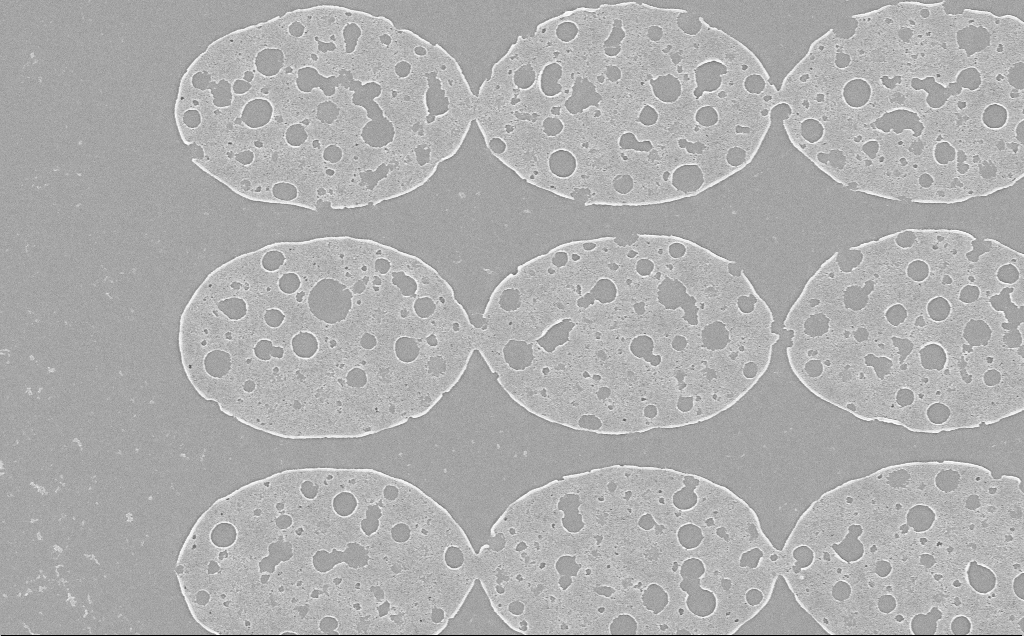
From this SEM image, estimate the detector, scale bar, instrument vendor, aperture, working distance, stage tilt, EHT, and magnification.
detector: InLens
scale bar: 2000 nm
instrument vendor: Zeiss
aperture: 30 µm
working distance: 6 mm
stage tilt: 0°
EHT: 5 kV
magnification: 11.19 K X